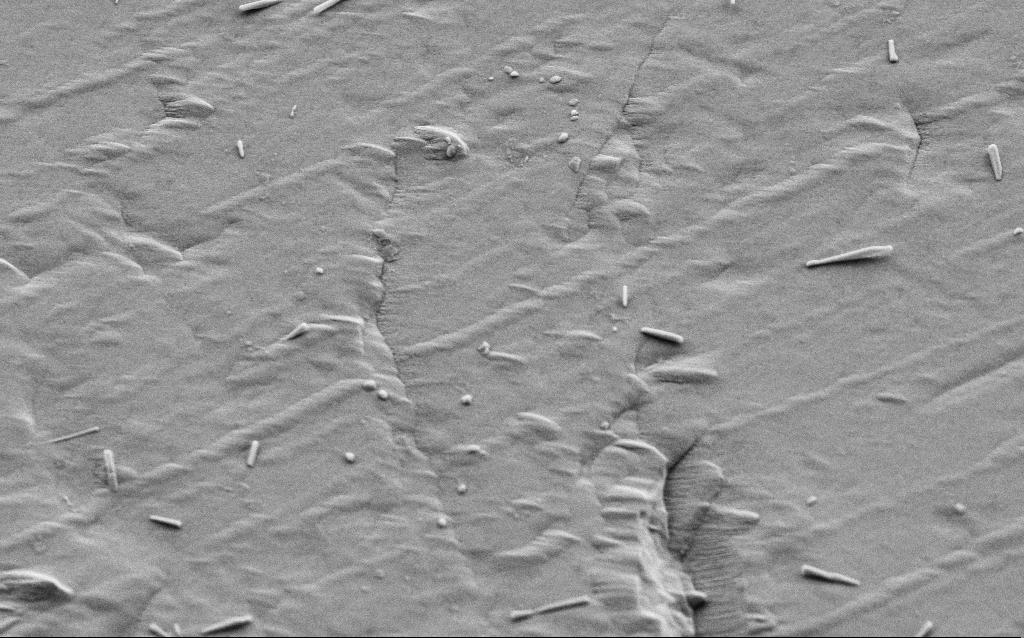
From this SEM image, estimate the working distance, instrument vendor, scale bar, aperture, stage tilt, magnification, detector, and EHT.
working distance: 4 mm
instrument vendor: Zeiss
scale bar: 2000 nm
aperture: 30 µm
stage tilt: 35°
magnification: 16.39 K X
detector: SE2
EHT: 5 kV